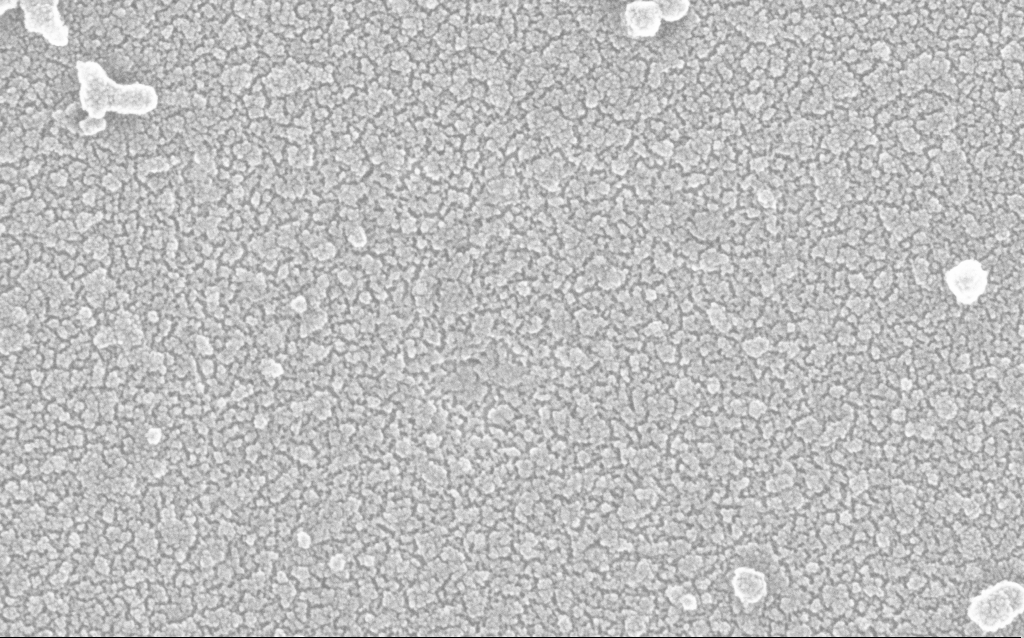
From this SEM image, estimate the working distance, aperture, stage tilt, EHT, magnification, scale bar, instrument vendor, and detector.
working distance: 1.9 mm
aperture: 30 µm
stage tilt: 0°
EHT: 20 kV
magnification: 300 K X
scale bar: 100 nm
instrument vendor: Zeiss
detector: InLens